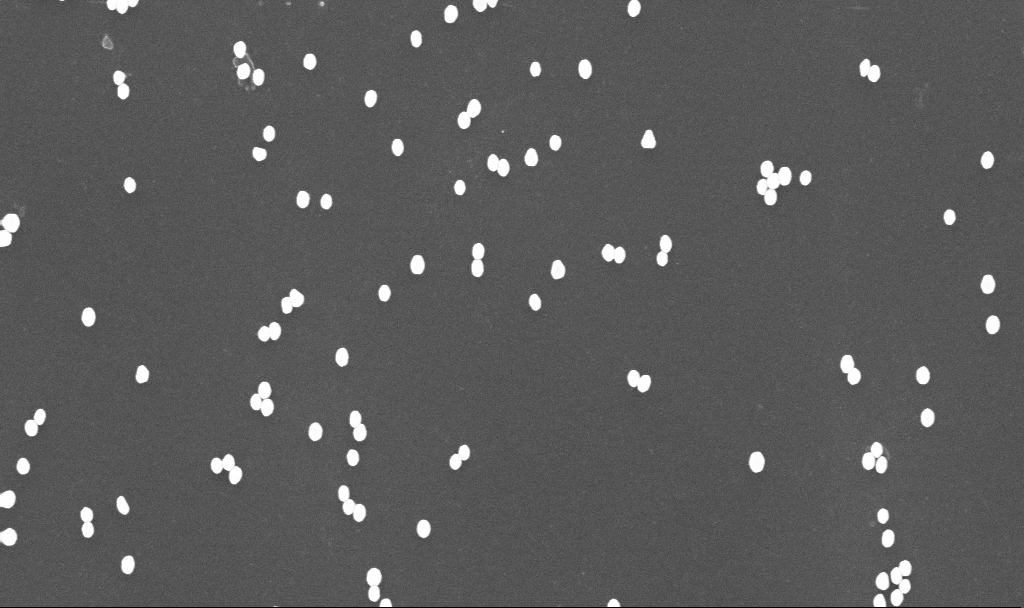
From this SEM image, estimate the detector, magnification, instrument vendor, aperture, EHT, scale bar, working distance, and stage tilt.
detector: InLens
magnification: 70 K X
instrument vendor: Zeiss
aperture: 30 µm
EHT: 10 kV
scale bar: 1000 nm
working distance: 3.4 mm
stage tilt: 0°